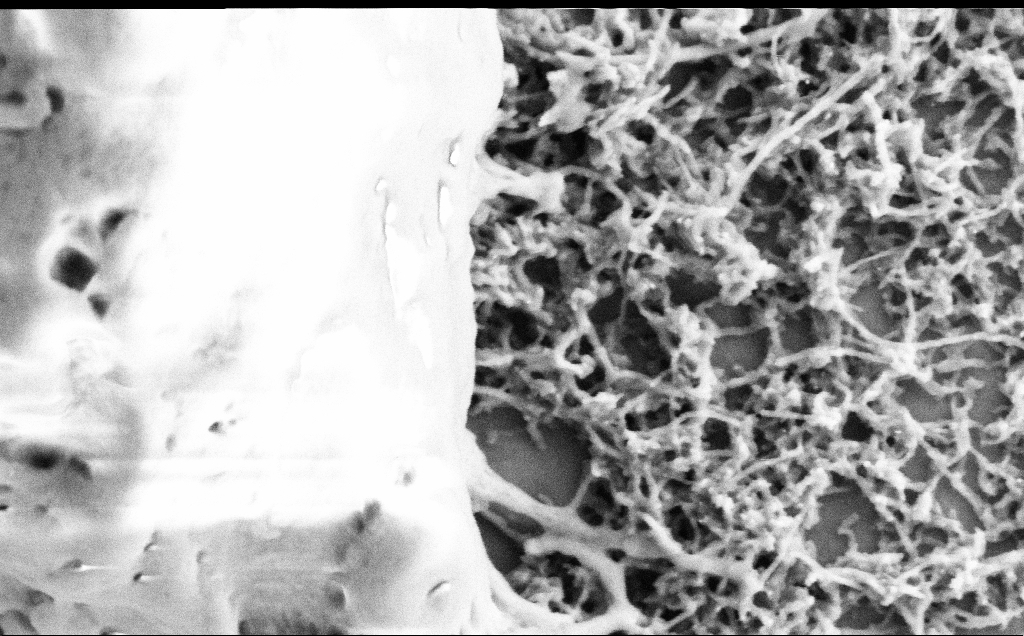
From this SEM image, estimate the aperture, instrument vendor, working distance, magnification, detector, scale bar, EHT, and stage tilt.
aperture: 30 µm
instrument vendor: Zeiss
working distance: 7.1 mm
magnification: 75 K X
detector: SE2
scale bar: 200 nm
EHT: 2 kV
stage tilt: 0°